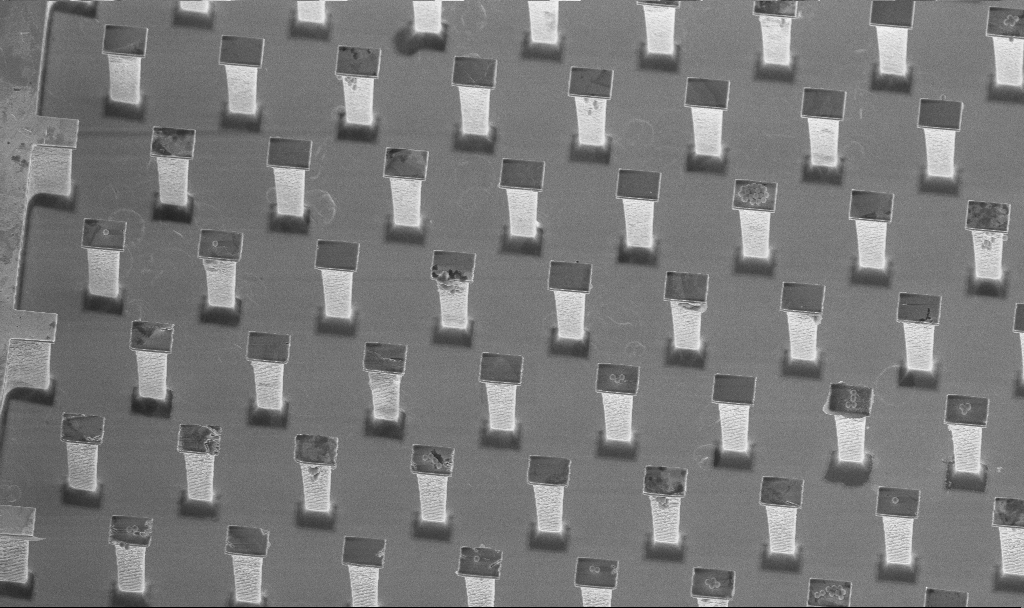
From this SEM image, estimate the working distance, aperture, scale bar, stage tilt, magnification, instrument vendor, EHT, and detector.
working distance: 5.8 mm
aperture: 30 µm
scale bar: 10000 nm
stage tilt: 20°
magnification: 3.57 K X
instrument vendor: Zeiss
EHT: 5 kV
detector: InLens